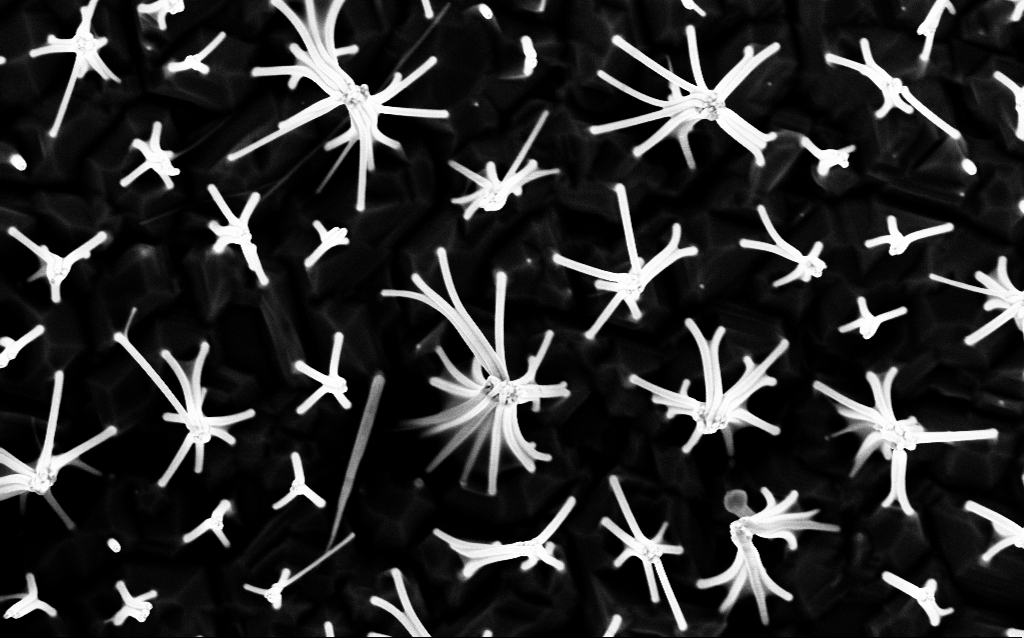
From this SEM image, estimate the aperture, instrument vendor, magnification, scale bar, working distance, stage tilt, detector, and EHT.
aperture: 30 µm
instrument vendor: Zeiss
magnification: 36.28 K X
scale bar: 1000 nm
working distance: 6.6 mm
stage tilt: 0°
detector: InLens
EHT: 5 kV